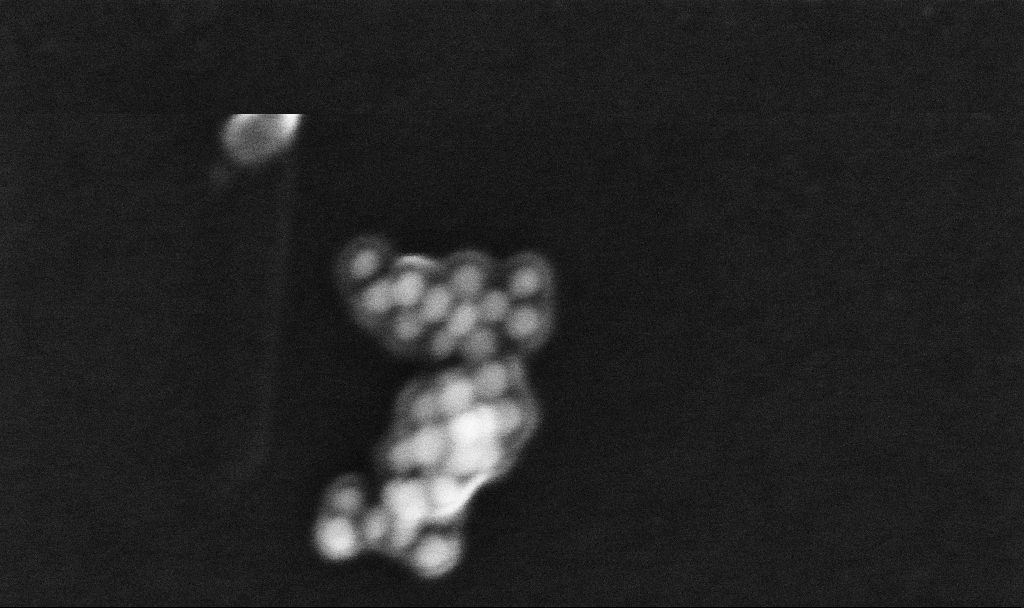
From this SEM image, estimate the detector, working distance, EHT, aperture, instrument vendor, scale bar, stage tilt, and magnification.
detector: InLens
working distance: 3.1 mm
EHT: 10 kV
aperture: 30 µm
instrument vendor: Zeiss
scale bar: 100 nm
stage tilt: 0°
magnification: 625.57 K X